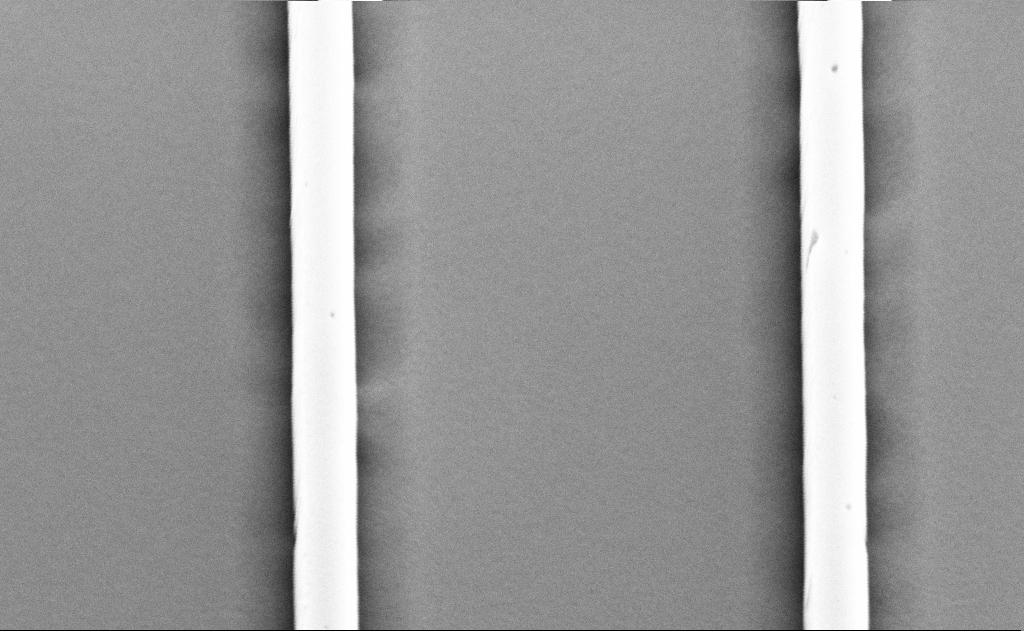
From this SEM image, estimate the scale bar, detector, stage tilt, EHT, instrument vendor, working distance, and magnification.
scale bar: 1000 nm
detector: SE2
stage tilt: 45°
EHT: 5 kV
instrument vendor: Zeiss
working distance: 11 mm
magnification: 46.82 K X